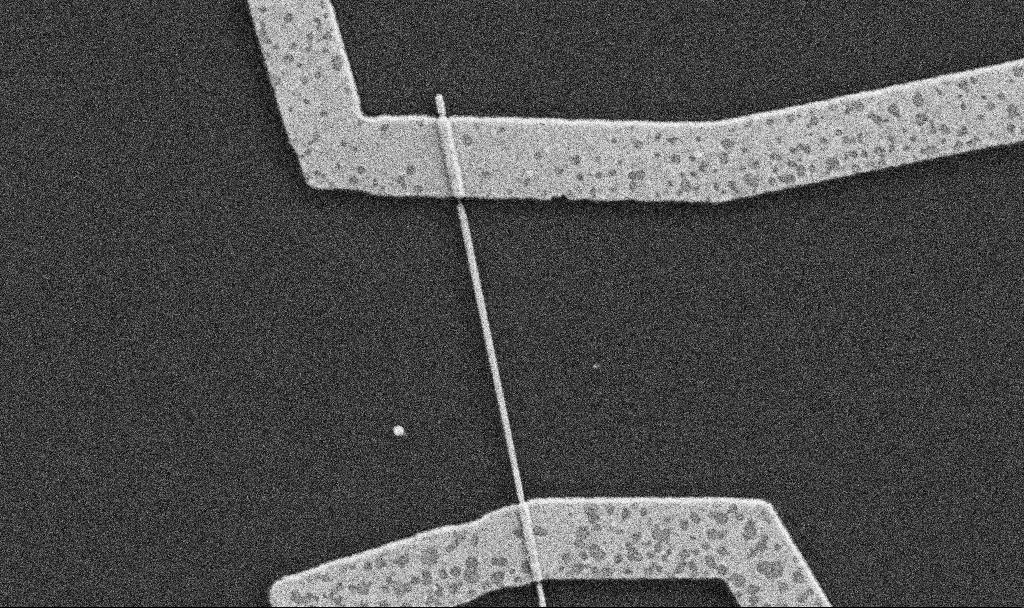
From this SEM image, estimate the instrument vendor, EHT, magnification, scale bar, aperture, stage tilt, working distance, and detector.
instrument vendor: Zeiss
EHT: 5 kV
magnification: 30 K X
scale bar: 1000 nm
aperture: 30 µm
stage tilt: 0°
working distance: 8.7 mm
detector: SE2